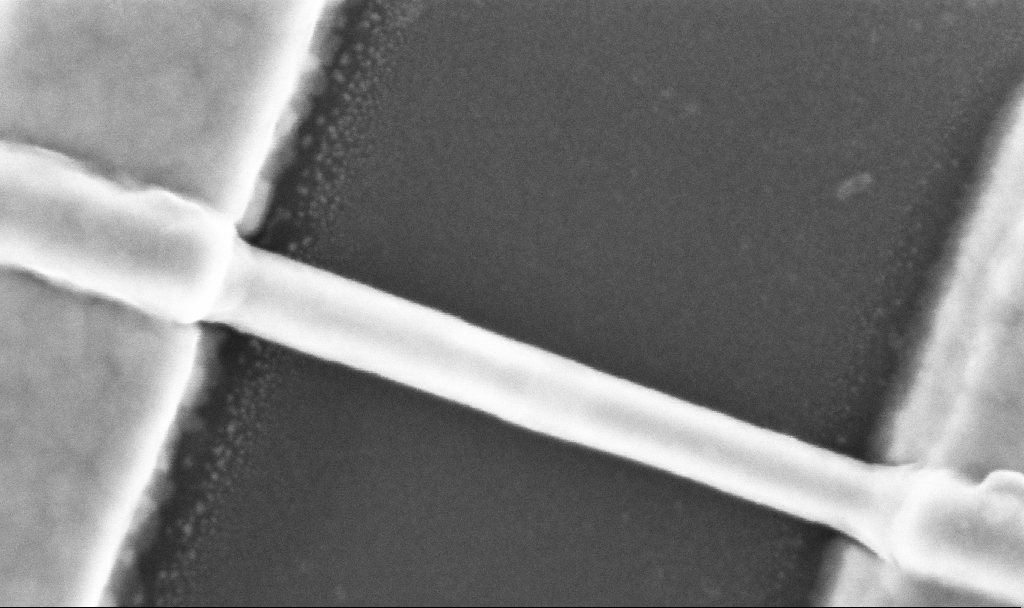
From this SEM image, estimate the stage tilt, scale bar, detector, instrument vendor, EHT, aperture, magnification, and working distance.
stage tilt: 0°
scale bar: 100 nm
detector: InLens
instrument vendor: Zeiss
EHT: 5 kV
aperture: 30 µm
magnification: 300 K X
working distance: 6.7 mm